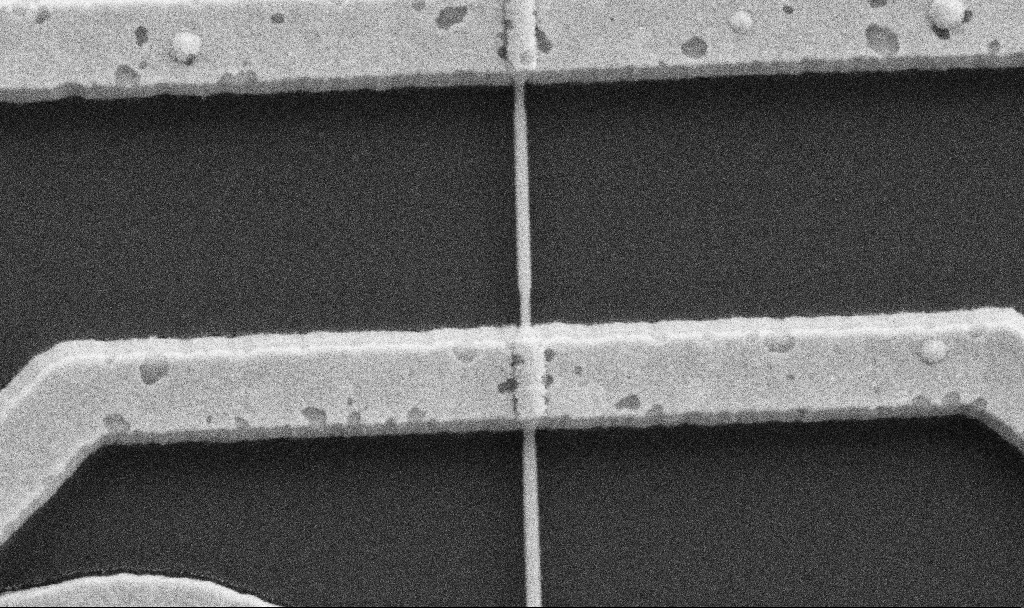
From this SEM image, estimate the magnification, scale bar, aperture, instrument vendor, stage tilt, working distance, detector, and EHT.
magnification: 67.04 K X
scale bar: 1000 nm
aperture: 30 µm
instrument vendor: Zeiss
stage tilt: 0°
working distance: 10.6 mm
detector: SE2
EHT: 5 kV